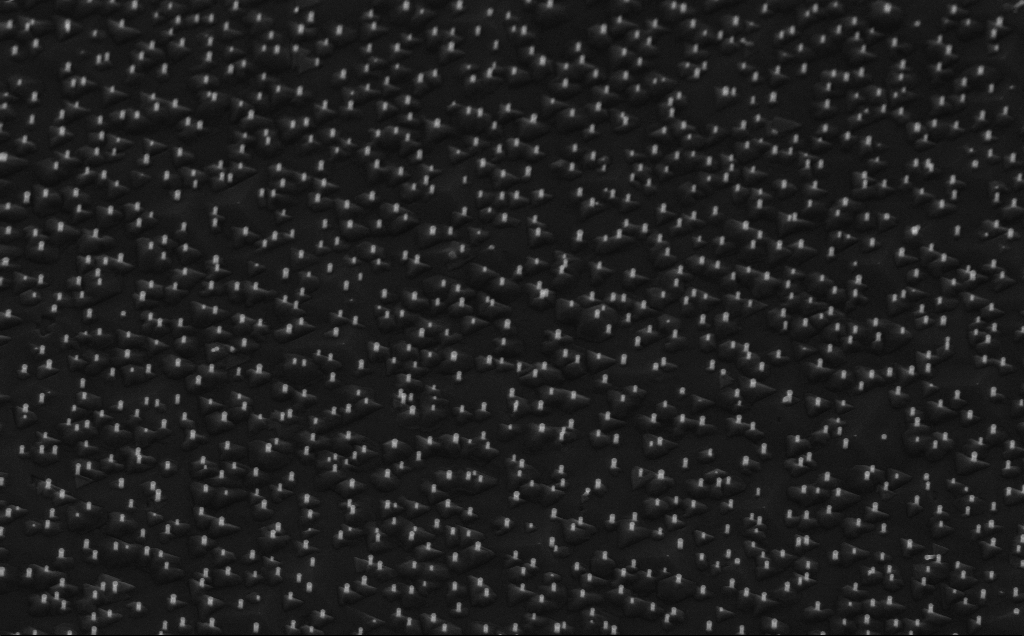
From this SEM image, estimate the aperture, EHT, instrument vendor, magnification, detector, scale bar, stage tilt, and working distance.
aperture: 30 µm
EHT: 10 kV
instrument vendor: Zeiss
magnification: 40 K X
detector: InLens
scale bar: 1000 nm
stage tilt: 45°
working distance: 6 mm